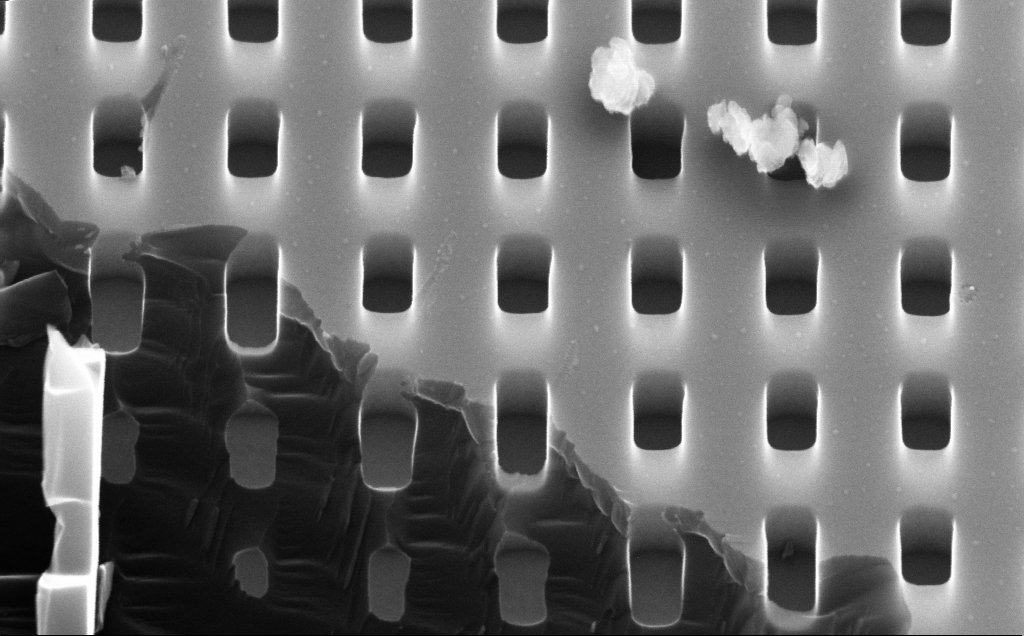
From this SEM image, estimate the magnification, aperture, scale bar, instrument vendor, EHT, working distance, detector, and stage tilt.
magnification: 100 K X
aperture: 30 µm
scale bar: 200 nm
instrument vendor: Zeiss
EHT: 10 kV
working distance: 5 mm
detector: InLens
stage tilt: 45°